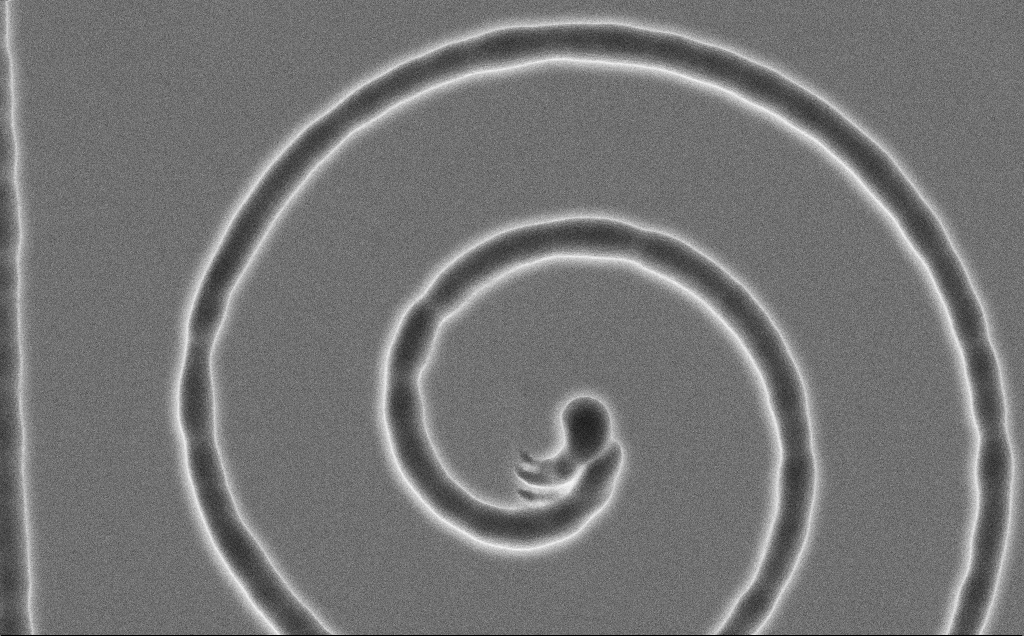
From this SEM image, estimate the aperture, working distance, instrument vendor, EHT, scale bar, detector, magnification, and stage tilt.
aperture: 30 µm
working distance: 13 mm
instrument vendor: Zeiss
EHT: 5 kV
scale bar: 2000 nm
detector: SE2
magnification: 11.39 K X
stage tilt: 0°